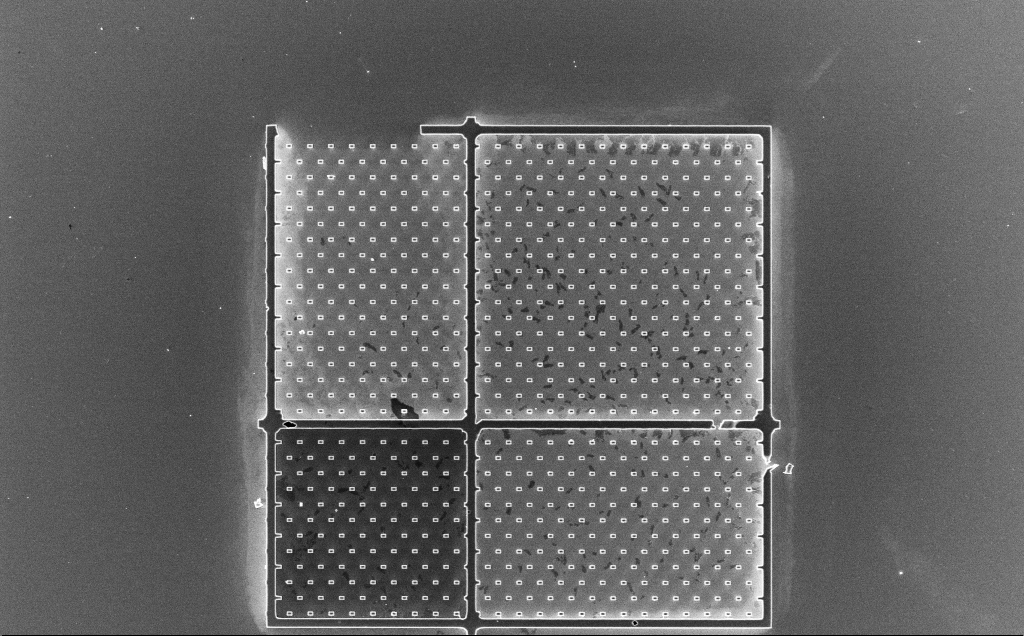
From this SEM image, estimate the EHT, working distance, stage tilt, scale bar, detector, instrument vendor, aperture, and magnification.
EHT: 10 kV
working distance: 3.2 mm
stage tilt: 0°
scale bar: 100000 nm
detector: InLens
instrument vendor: Zeiss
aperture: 30 µm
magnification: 0.637 K X